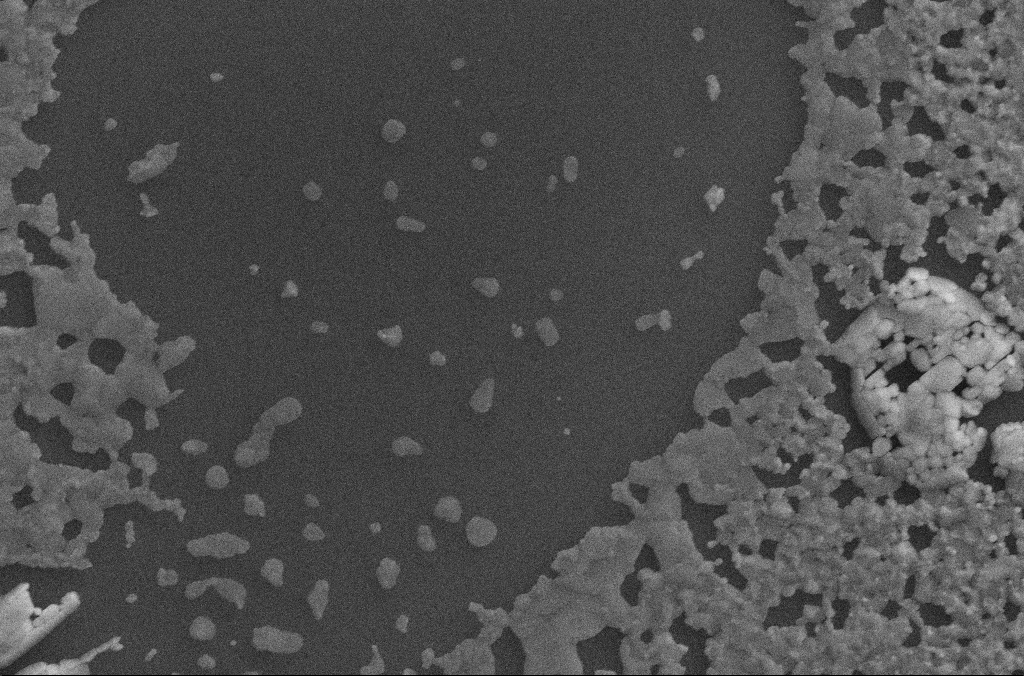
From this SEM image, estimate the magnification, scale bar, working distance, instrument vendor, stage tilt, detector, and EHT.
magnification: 5 K X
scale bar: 10000 nm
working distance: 2.8 mm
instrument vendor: Zeiss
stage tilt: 20°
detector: SE2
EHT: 20 kV